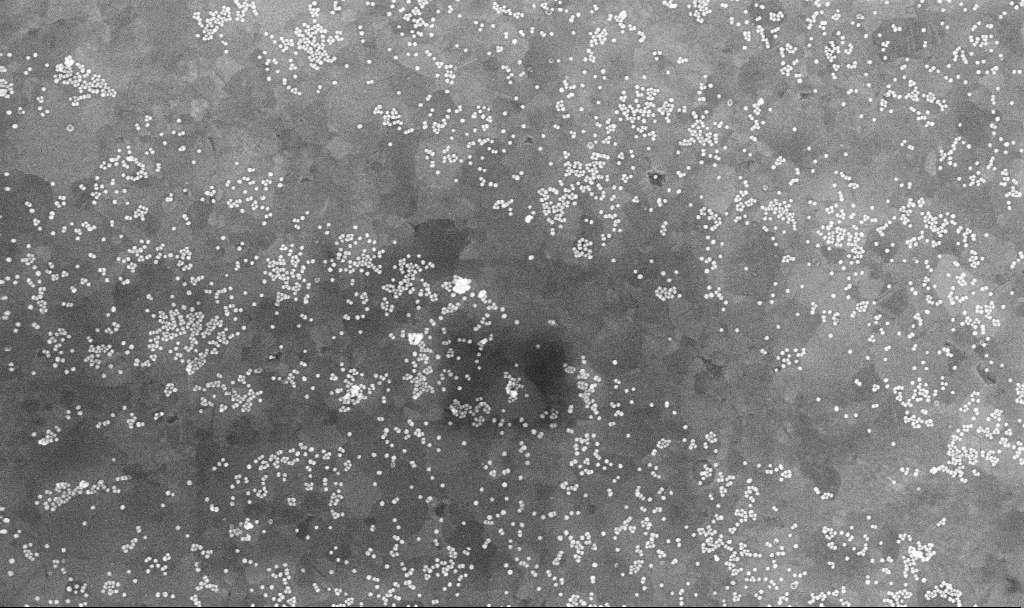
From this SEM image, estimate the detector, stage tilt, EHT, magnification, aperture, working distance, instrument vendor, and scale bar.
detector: InLens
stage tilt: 0°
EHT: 10 kV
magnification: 70 K X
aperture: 30 µm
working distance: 3.7 mm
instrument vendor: Zeiss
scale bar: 1000 nm